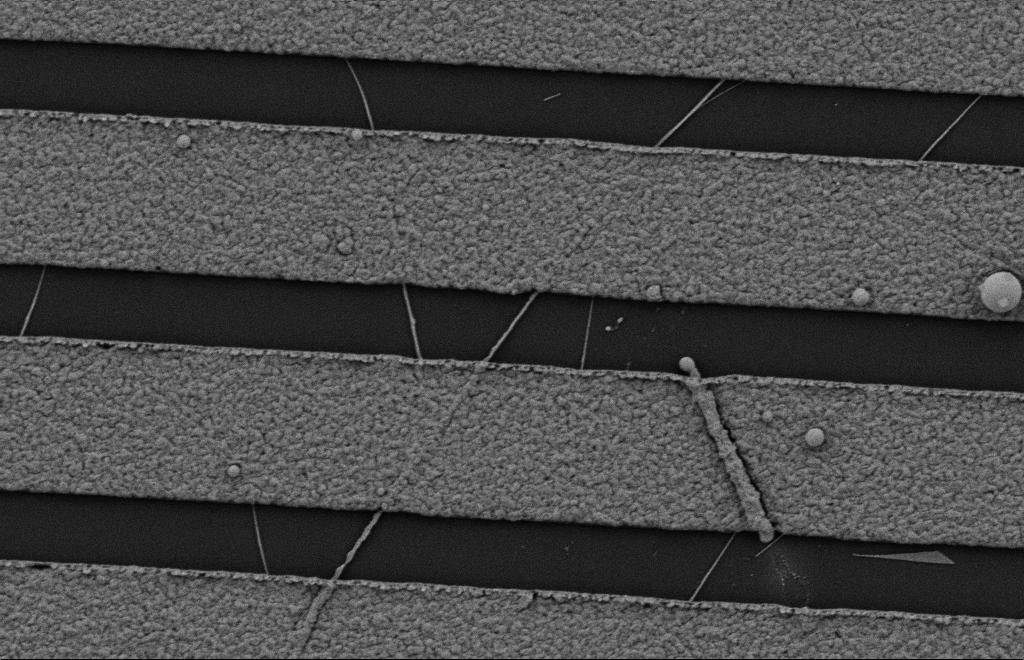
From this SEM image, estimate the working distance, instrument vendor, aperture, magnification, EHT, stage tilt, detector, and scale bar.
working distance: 10 mm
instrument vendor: Zeiss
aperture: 20 µm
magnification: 20.75 K X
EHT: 2 kV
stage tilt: -0.3°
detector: SE2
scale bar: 2000 nm